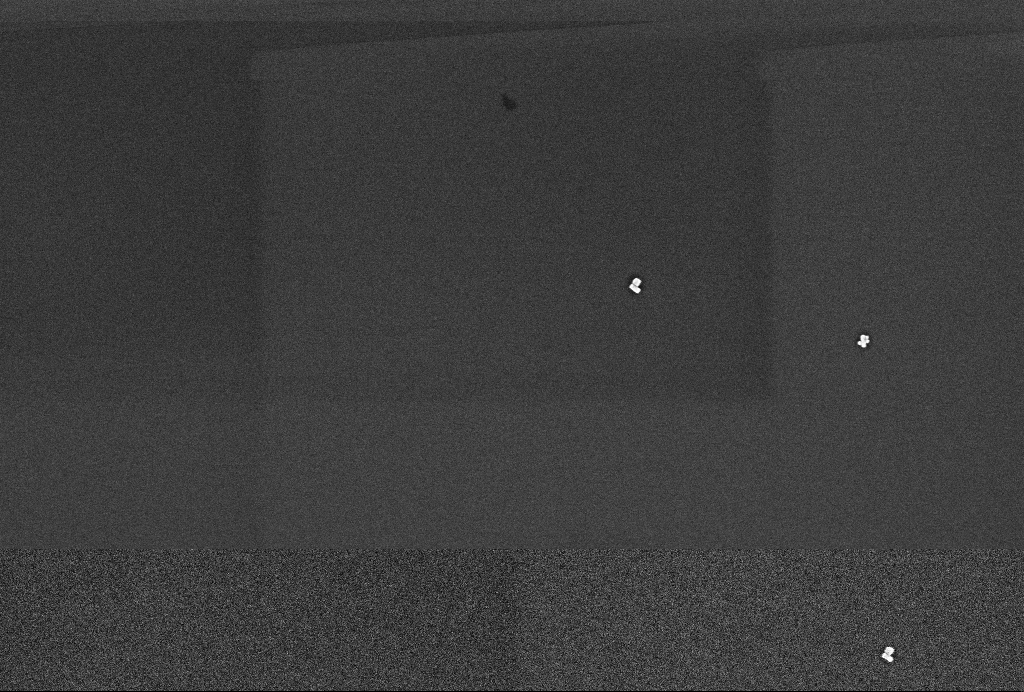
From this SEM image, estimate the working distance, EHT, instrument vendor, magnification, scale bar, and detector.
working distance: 3.3 mm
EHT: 2 kV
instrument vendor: Zeiss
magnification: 60 K X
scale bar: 1000 nm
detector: InLens